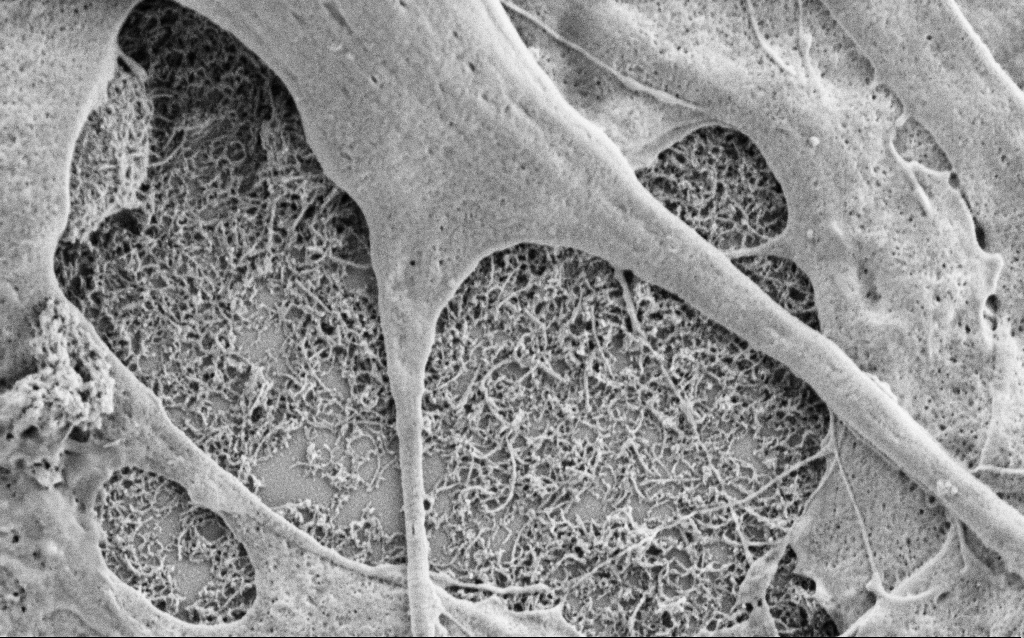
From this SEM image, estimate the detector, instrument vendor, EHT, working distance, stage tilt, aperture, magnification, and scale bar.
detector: SE2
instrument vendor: Zeiss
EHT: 1.5 kV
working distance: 6.8 mm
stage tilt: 0°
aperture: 30 µm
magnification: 25 K X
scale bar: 2000 nm